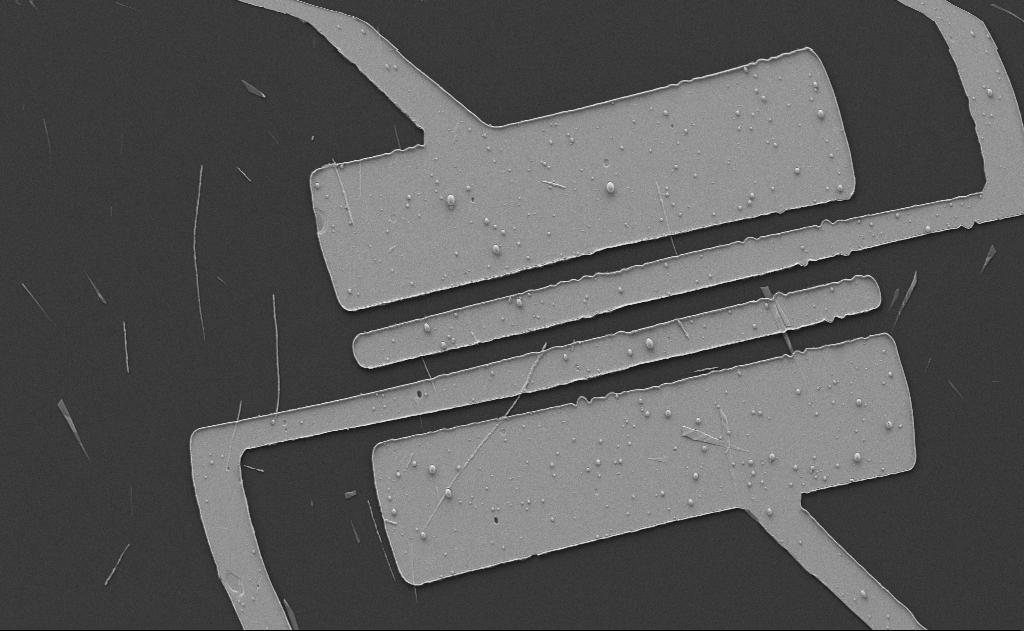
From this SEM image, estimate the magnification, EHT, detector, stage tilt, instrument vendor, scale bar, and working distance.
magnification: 3.38 K X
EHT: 5 kV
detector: SE2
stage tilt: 0°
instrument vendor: Zeiss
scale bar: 10000 nm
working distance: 10 mm